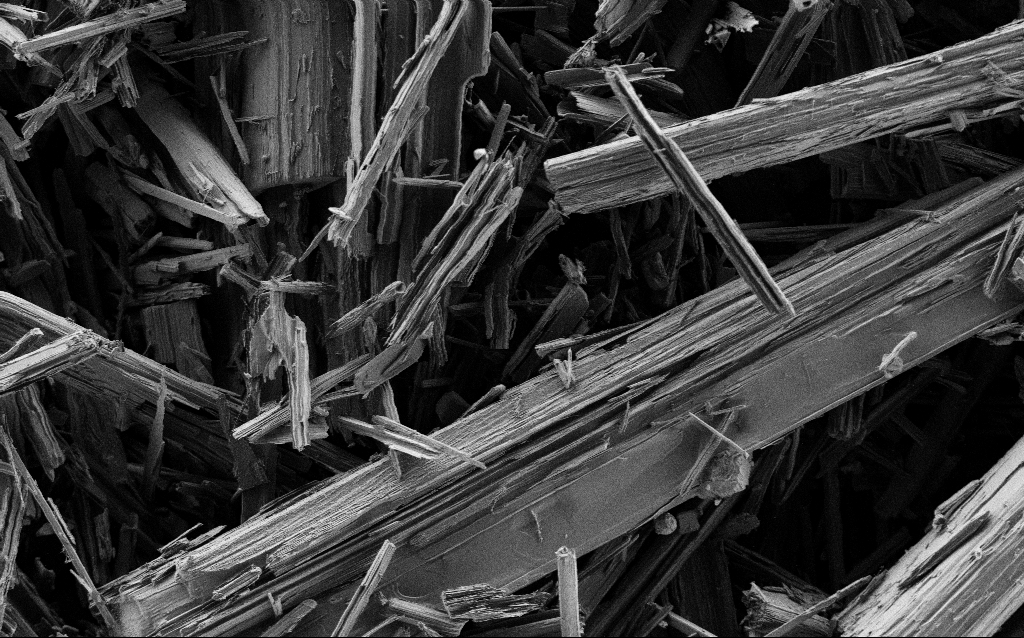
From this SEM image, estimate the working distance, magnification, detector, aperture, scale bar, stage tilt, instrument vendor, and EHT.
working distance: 4.3 mm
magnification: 2.5 K X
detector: SE2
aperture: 30 µm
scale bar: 10000 nm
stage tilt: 0°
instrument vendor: Zeiss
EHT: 0.8 kV